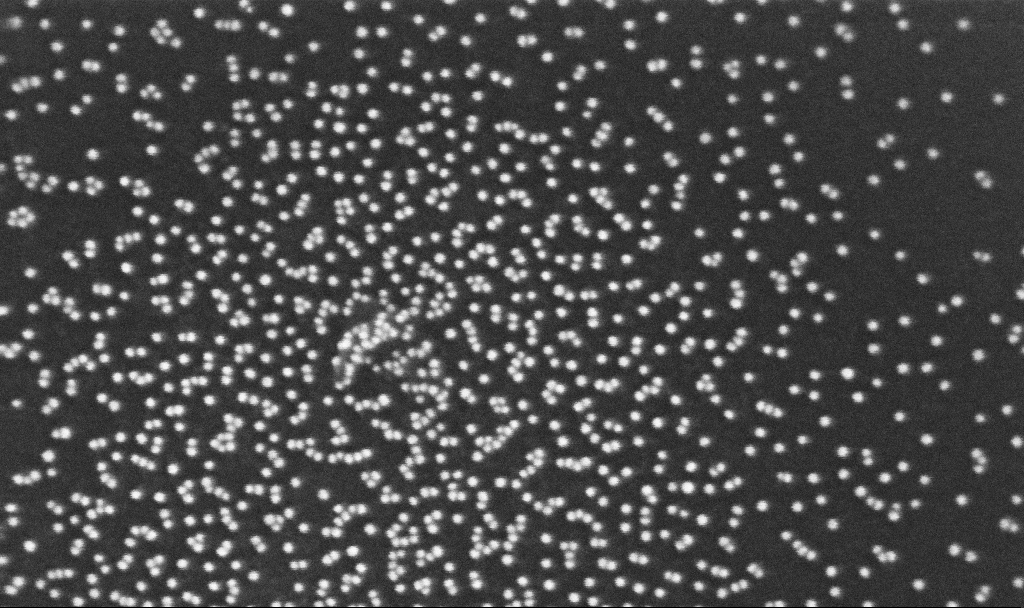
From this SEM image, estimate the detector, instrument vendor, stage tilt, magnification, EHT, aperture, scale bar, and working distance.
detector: InLens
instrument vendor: Zeiss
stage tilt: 0°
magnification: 350 K X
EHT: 10 kV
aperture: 30 µm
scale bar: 200 nm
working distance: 3.2 mm